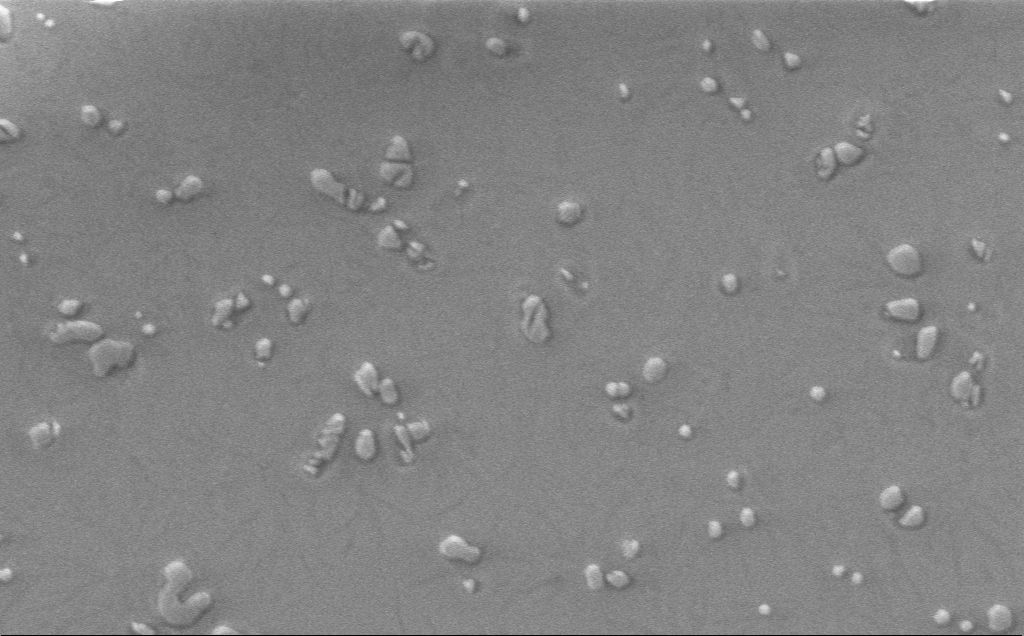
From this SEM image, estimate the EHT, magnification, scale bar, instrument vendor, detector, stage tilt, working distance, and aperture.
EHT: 1 kV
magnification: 5.54 K X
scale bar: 10000 nm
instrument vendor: Zeiss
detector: InLens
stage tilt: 0°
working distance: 4 mm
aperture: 30 µm